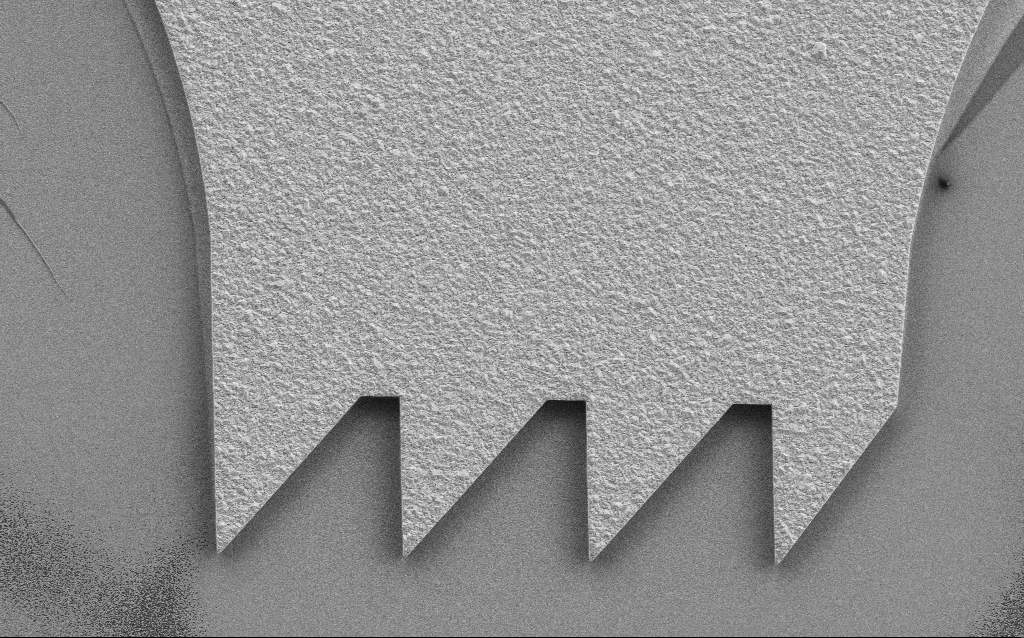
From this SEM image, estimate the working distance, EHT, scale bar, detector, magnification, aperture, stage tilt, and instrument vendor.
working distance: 6.9 mm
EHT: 3 kV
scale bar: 10000 nm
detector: SE2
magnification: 6 K X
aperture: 30 µm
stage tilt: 0°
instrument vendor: Zeiss